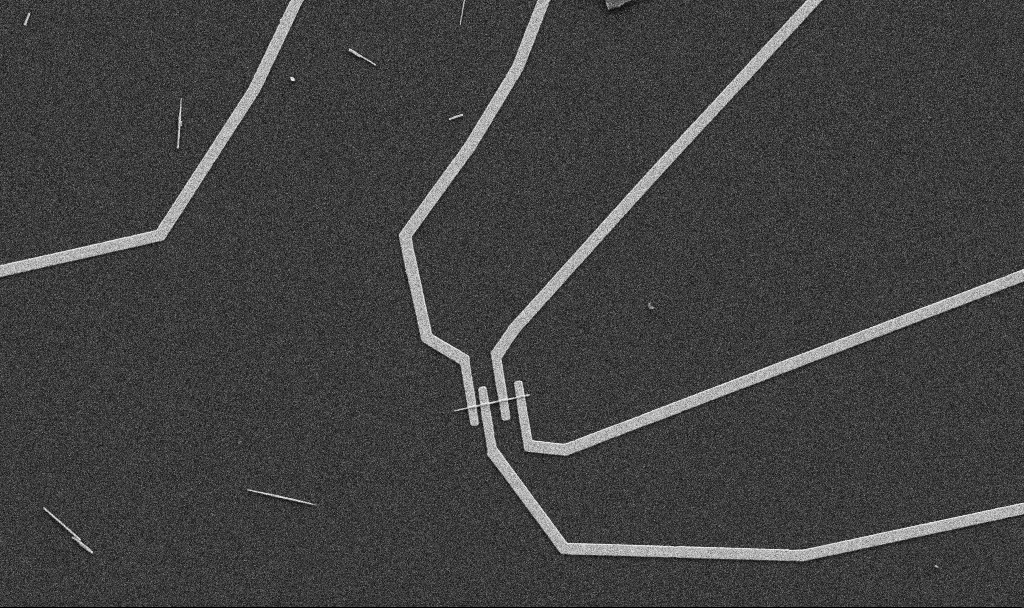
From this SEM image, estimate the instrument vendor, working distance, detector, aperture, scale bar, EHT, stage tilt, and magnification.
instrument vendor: Zeiss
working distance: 10.7 mm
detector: SE2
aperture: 30 µm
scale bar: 10000 nm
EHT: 5 kV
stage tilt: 0°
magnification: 5 K X